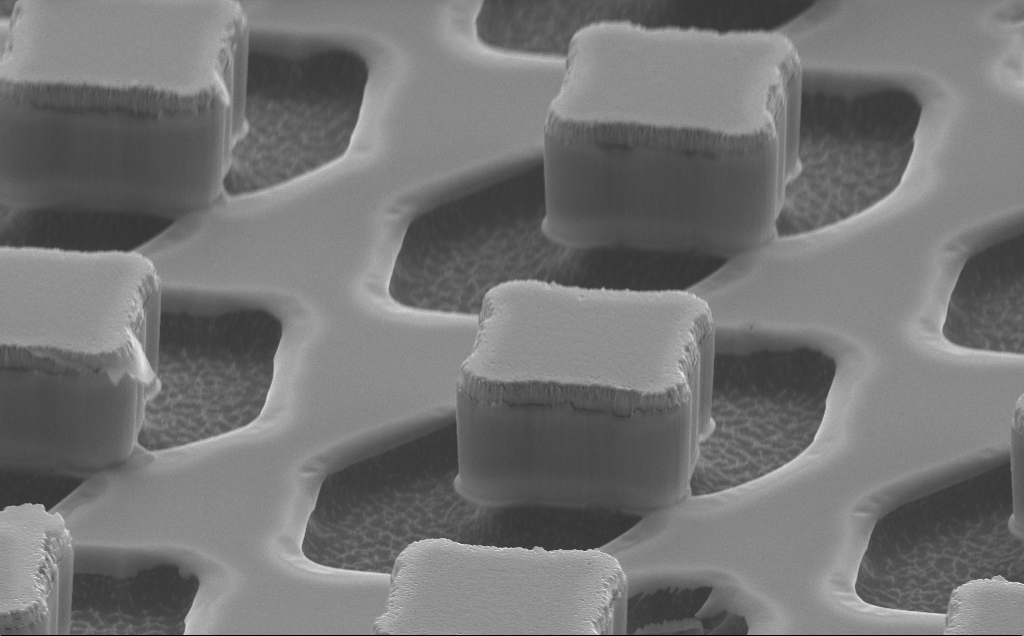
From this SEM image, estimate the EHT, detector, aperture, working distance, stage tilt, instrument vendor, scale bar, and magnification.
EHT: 10 kV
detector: SE2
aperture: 30 µm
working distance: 12 mm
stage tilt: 61.7°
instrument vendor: Zeiss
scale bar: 2000 nm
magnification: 10.27 K X